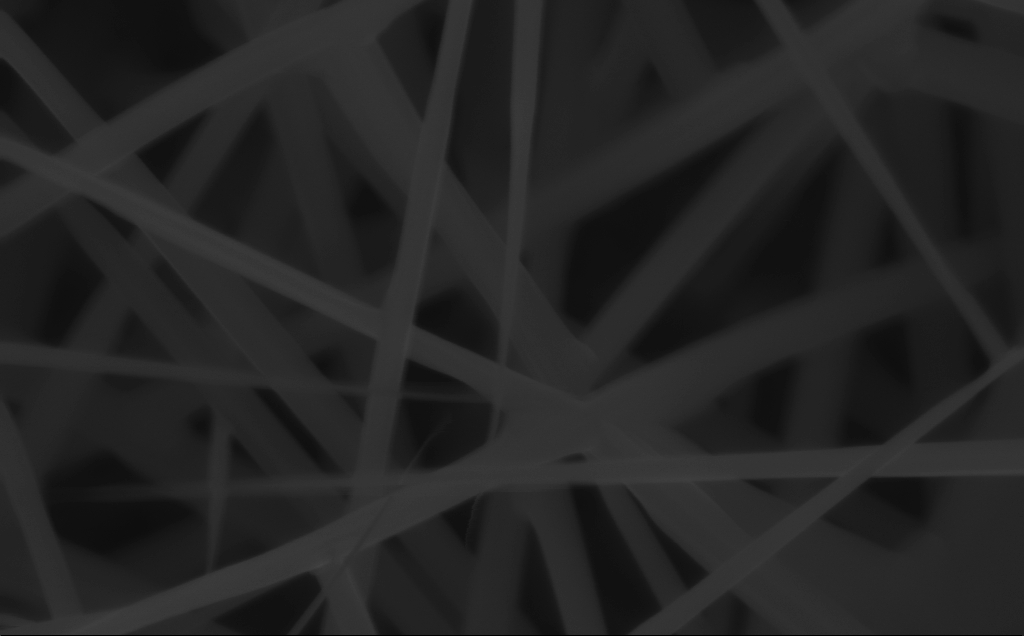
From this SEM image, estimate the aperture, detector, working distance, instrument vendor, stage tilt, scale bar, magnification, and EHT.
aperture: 30 µm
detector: InLens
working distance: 4 mm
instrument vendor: Zeiss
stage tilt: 0°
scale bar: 200 nm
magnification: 150 K X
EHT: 10 kV